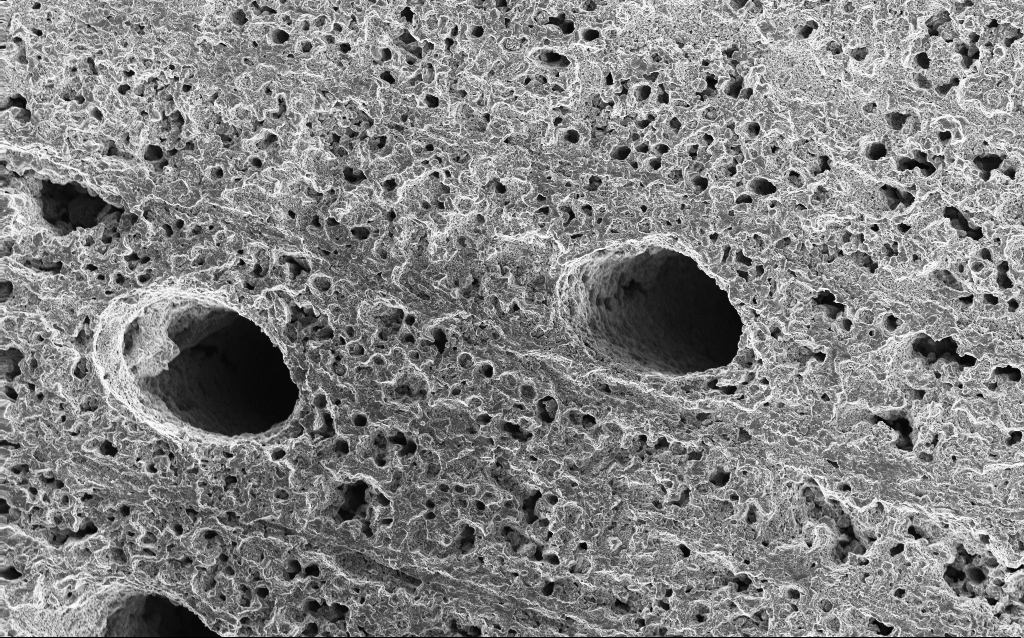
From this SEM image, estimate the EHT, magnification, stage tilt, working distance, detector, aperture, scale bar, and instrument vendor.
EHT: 10 kV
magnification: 0.5 K X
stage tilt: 0°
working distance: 3 mm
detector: InLens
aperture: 30 µm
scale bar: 100000 nm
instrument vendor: Zeiss